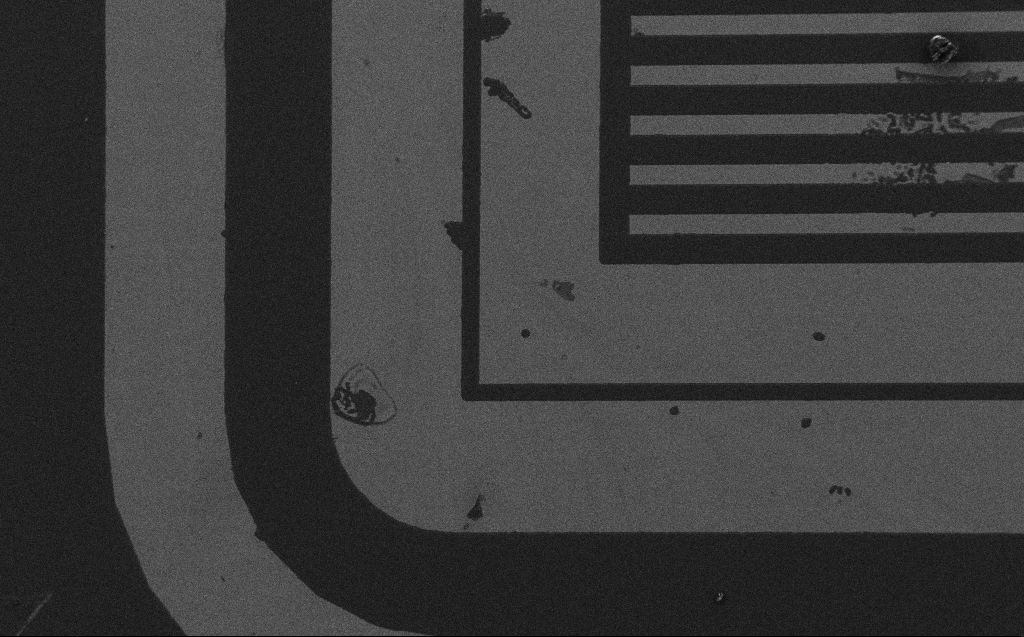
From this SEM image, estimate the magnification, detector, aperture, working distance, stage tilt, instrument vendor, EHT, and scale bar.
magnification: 0.458 K X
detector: SE2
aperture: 30 µm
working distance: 4 mm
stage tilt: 0°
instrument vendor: Zeiss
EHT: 1.2 kV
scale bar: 100000 nm